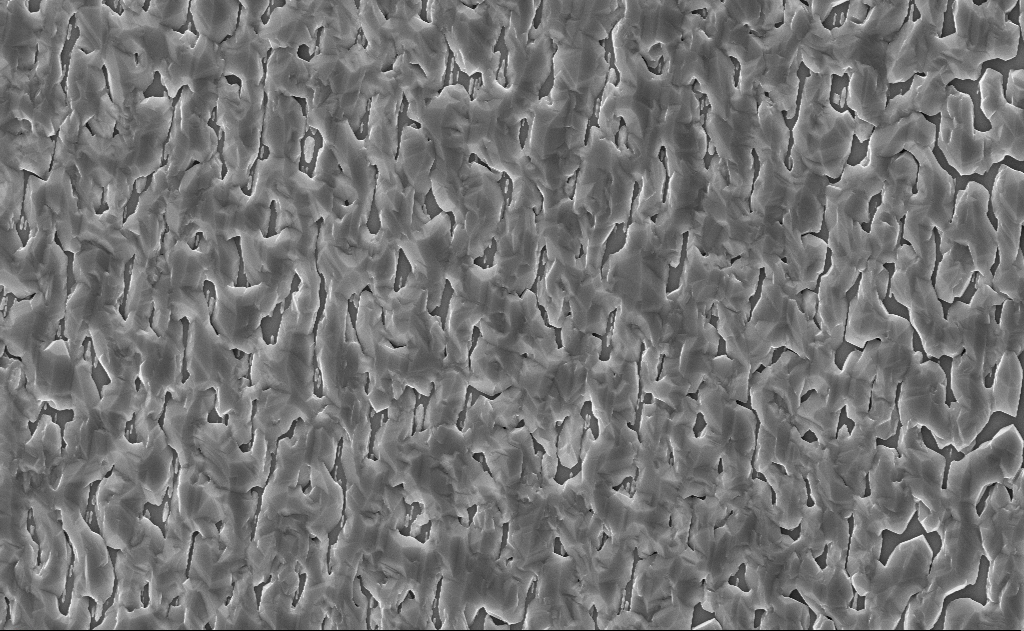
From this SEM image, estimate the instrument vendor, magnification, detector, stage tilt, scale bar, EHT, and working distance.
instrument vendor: Zeiss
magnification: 10 K X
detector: InLens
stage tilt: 0°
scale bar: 2000 nm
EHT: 10 kV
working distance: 14 mm